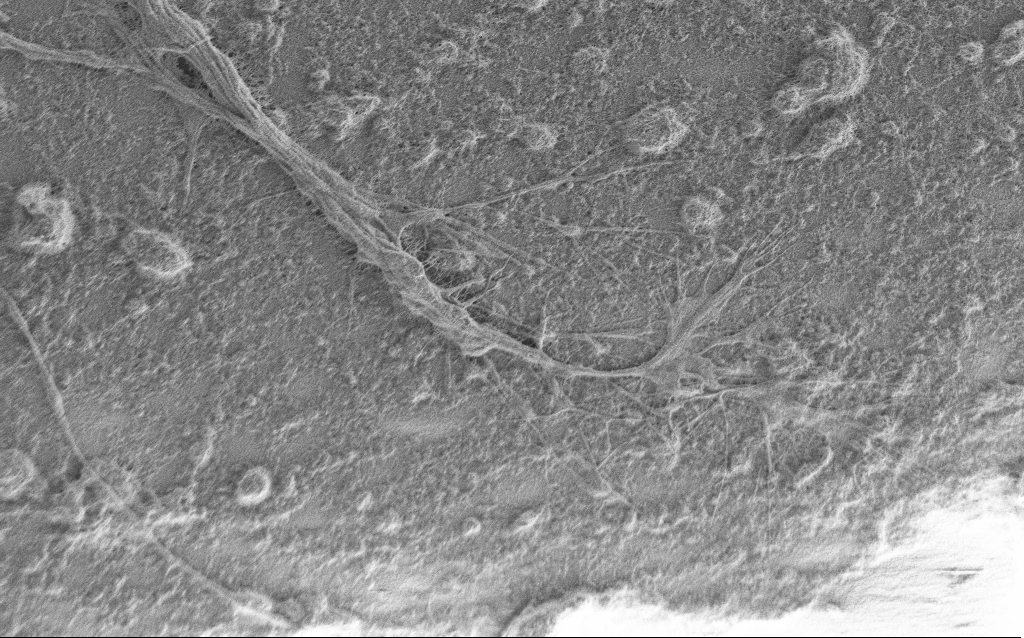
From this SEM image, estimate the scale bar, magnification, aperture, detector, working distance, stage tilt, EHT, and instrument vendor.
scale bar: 10000 nm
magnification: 6.5 K X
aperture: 30 µm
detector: SE2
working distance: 6 mm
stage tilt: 0°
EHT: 1 kV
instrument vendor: Zeiss